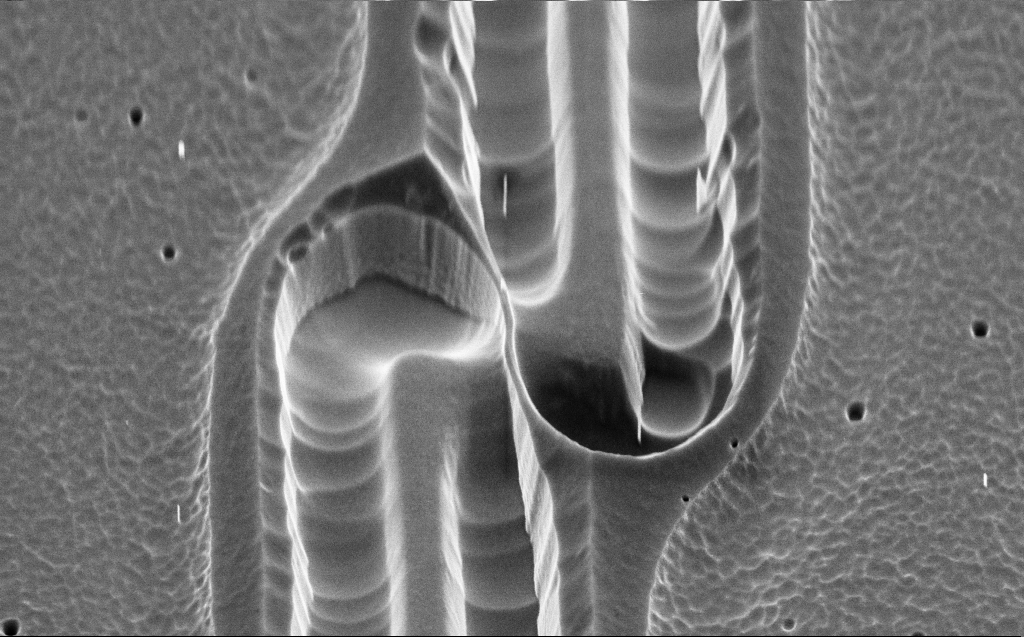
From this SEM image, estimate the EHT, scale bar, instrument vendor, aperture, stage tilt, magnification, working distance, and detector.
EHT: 3 kV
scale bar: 2000 nm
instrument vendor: Zeiss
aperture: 30 µm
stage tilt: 45°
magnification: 25.26 K X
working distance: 3 mm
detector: InLens